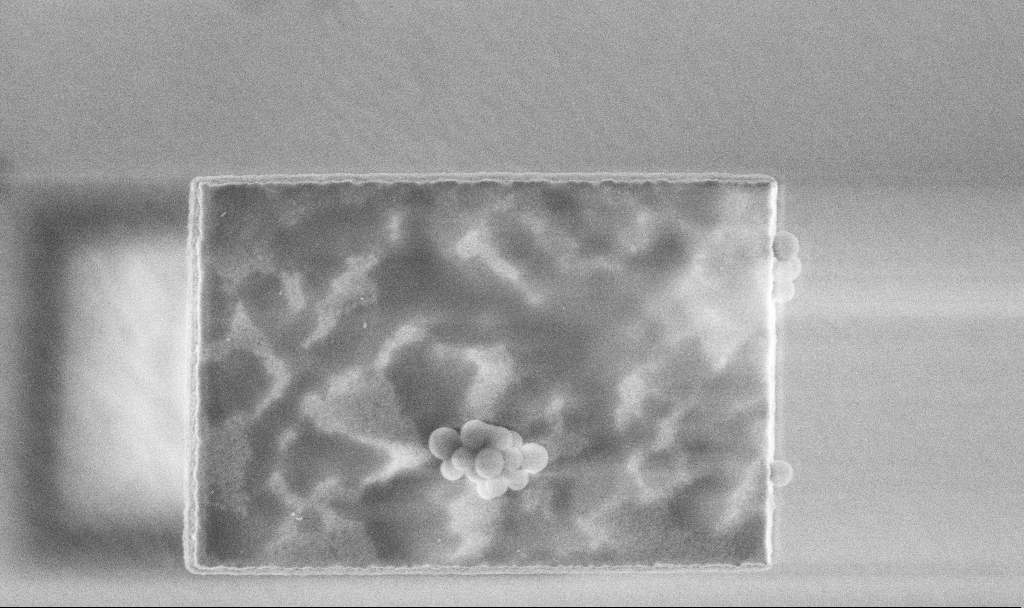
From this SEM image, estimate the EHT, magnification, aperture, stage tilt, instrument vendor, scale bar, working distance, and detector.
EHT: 3 kV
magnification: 48.27 K X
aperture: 30 µm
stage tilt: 0°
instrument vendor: Zeiss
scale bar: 1000 nm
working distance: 3.3 mm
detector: InLens